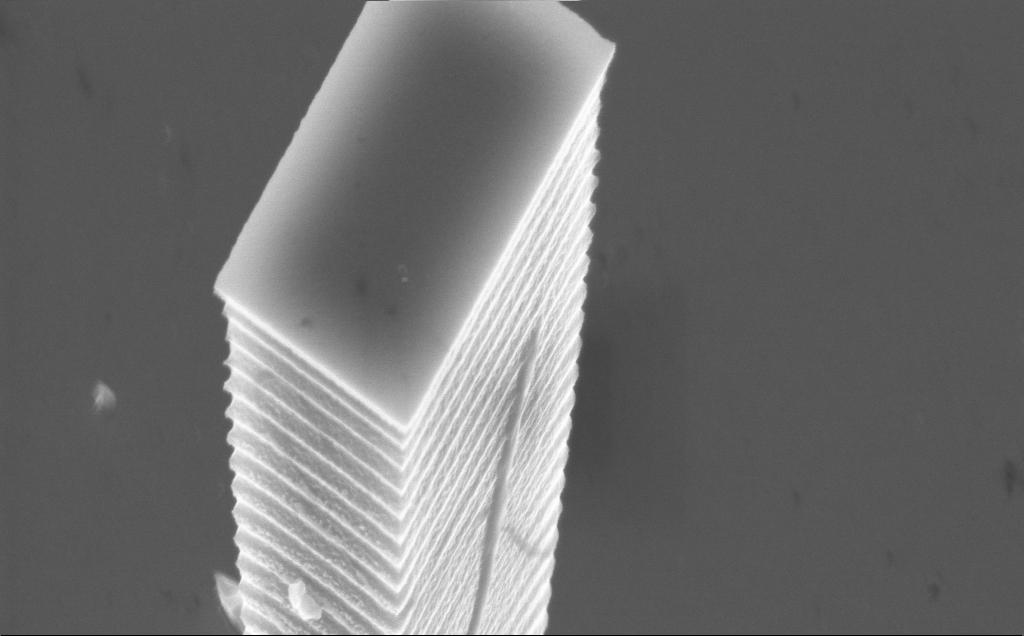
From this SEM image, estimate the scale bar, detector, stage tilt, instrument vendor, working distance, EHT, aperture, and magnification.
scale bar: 1000 nm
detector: InLens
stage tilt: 36.9°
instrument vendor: Zeiss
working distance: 4 mm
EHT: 10 kV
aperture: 30 µm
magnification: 29.48 K X